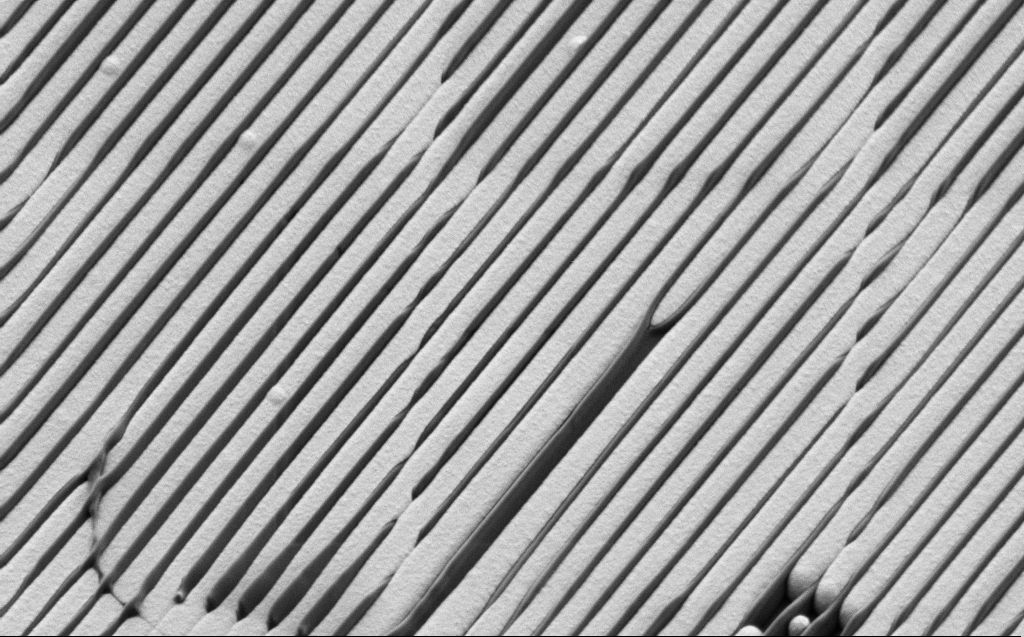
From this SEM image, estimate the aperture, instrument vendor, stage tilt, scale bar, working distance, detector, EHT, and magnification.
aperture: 30 µm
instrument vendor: Zeiss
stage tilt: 30°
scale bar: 2000 nm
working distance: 6 mm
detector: SE2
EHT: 1.1 kV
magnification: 9.77 K X